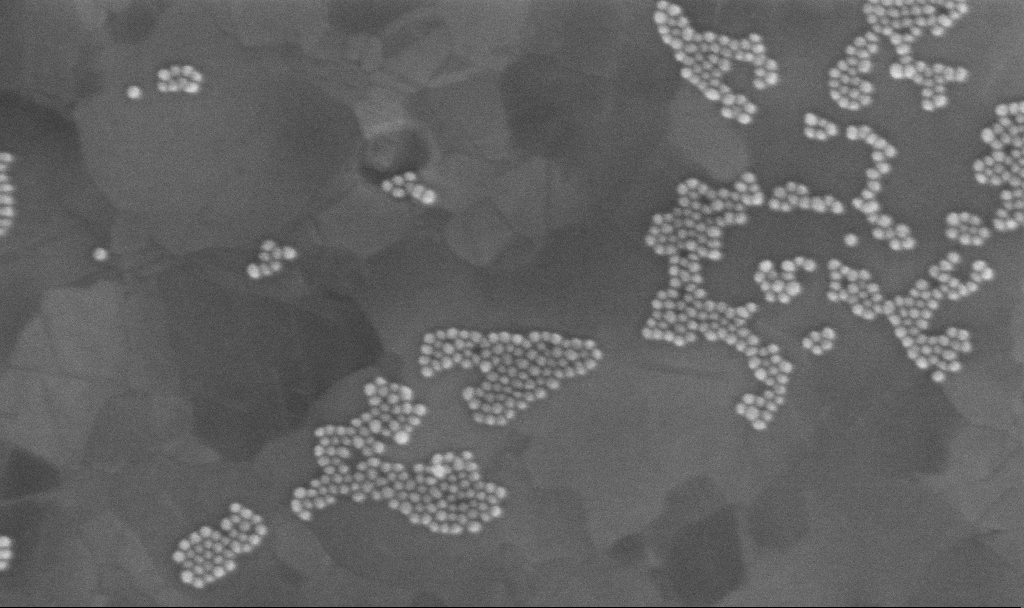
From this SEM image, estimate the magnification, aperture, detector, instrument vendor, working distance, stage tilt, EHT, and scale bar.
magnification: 250 K X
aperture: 30 µm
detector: InLens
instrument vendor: Zeiss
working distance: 3.4 mm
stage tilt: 0°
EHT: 10 kV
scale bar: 200 nm